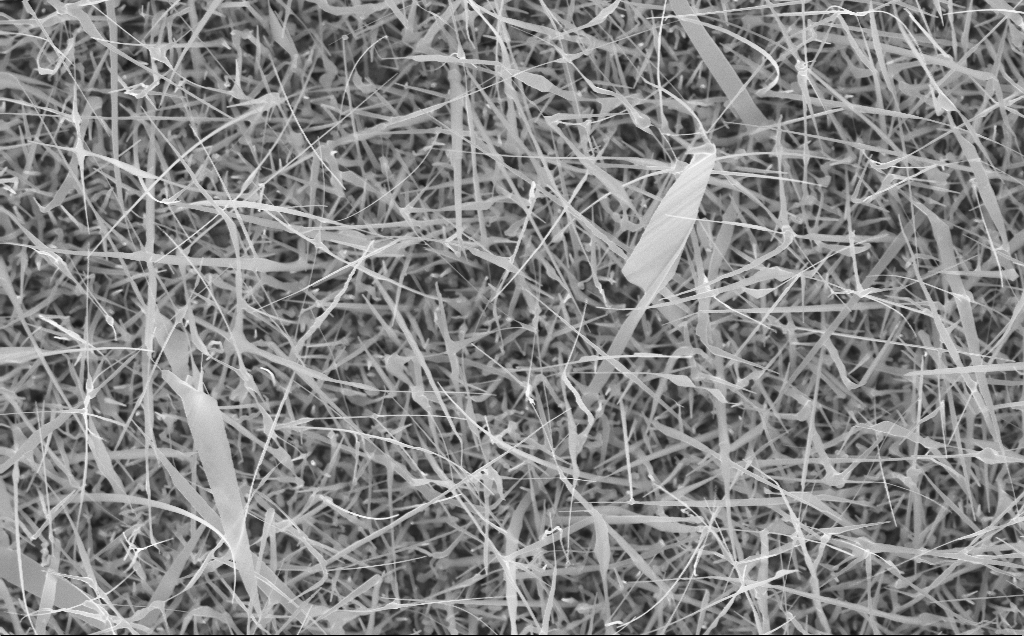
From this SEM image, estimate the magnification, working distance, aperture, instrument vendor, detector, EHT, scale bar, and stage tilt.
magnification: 20 K X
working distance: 4 mm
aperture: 30 µm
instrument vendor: Zeiss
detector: InLens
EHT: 10 kV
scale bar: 1000 nm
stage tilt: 0°